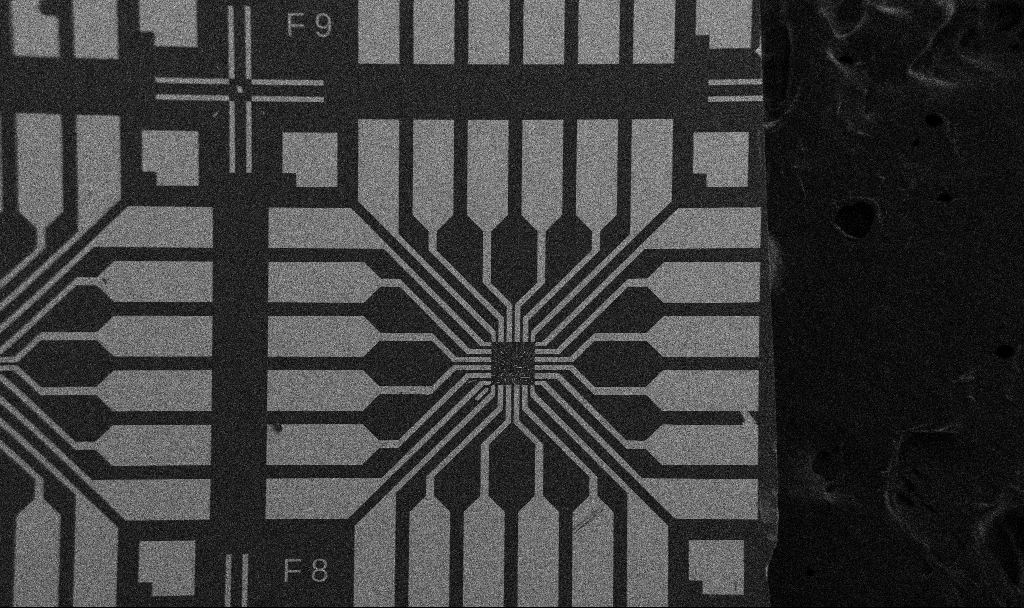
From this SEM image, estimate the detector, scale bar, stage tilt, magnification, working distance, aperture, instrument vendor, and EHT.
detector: SE2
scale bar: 200000 nm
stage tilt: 0°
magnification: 0.1 K X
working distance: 10.7 mm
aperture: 30 µm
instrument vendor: Zeiss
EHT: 5 kV